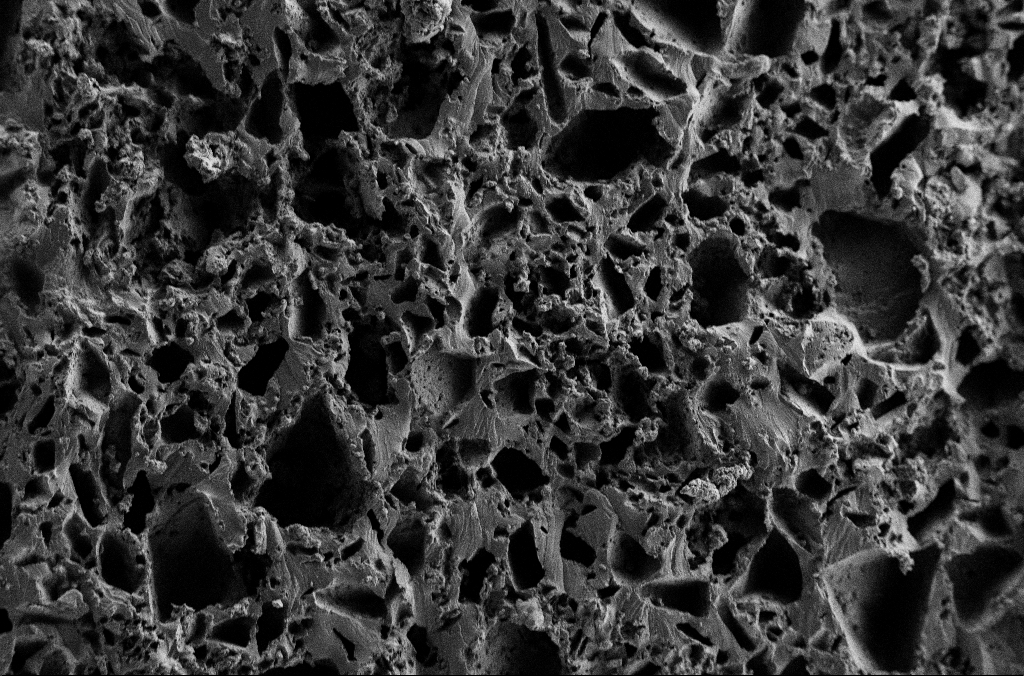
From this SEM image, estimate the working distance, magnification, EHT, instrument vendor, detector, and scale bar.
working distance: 2.9 mm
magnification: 0.25 K X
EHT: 2 kV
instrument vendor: Zeiss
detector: SE2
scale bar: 100000 nm